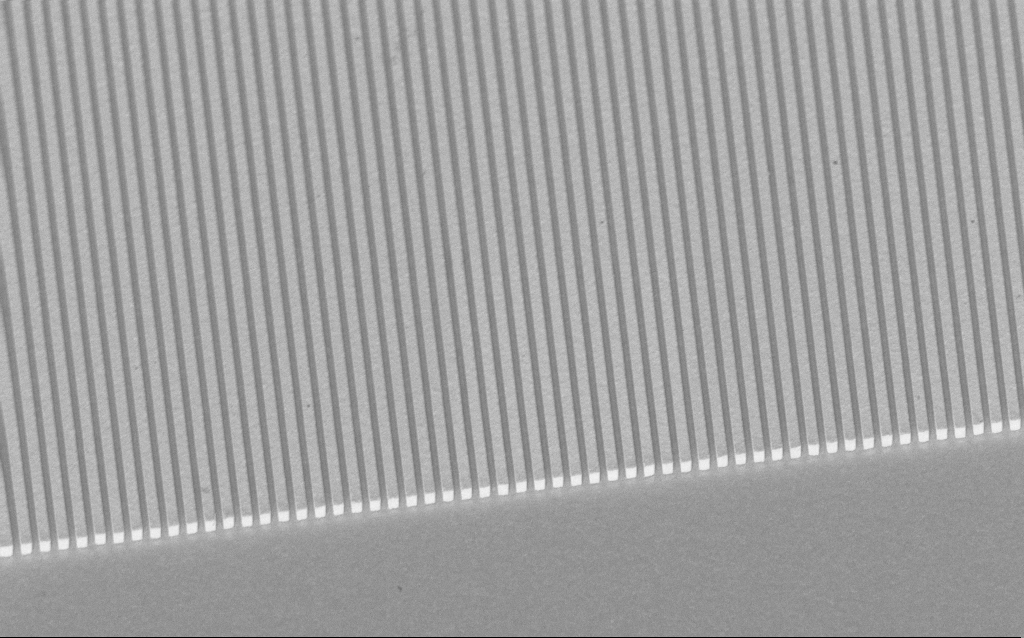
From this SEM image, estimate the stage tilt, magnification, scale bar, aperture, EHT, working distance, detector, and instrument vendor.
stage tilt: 45°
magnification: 10.96 K X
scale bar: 2000 nm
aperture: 30 µm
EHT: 1.5 kV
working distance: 8.6 mm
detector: InLens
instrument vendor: Zeiss